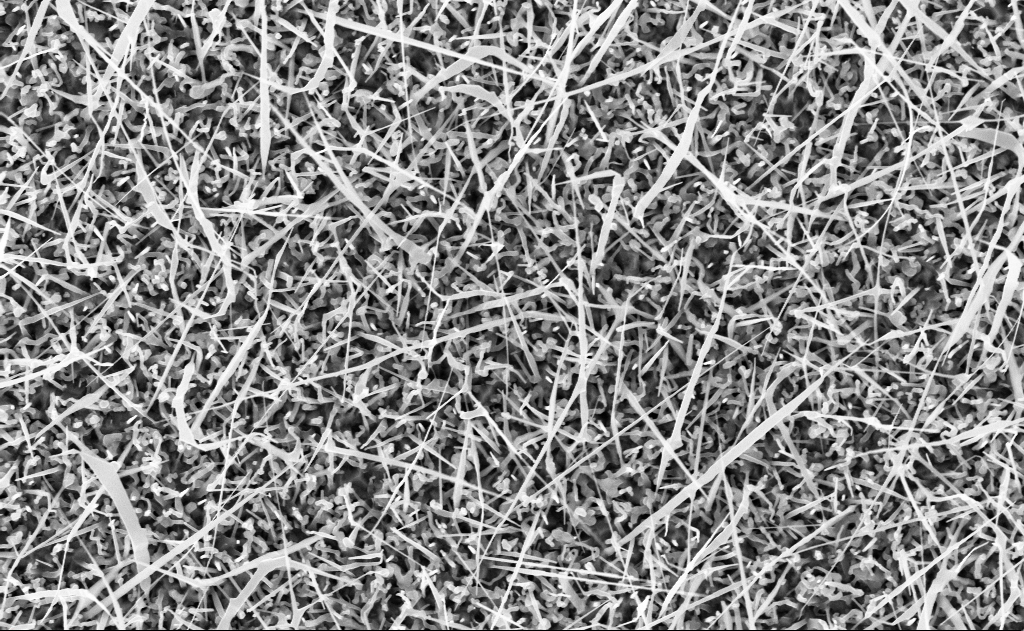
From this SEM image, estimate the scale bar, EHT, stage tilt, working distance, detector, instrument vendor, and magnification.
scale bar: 2000 nm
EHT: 10 kV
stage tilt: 0°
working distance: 10 mm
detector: InLens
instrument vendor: Zeiss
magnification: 20 K X